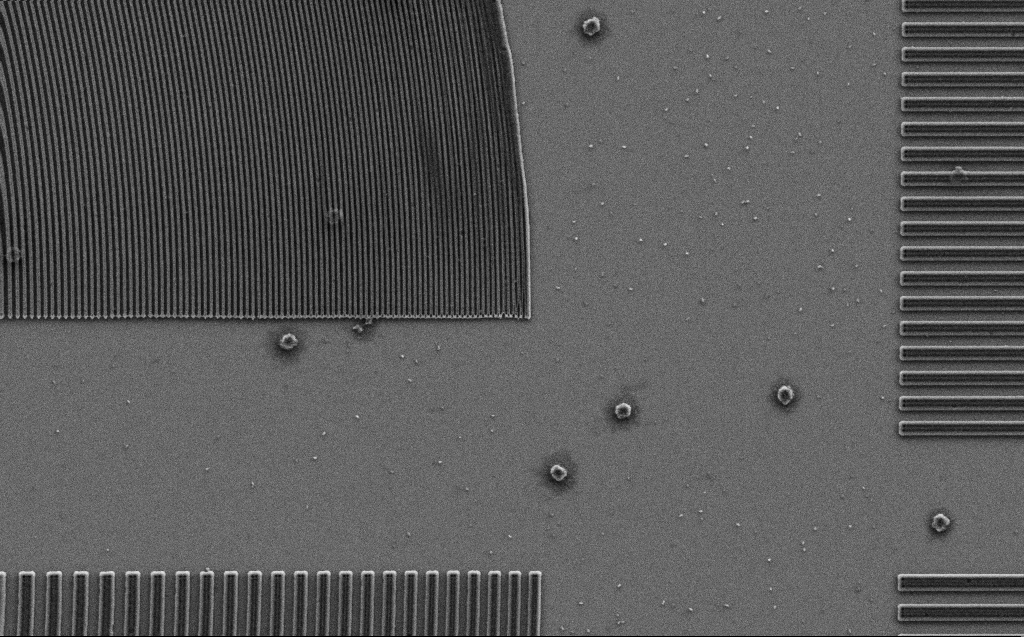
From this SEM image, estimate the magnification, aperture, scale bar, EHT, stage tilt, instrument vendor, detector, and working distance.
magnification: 9.29 K X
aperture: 30 µm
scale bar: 2000 nm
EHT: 3 kV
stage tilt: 0°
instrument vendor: Zeiss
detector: SE2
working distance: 6 mm